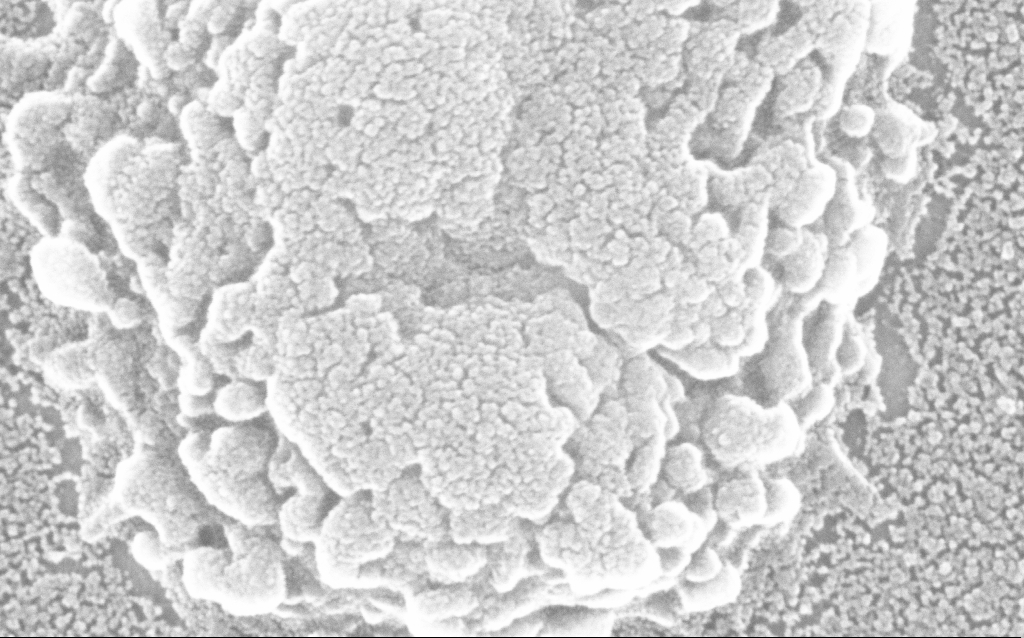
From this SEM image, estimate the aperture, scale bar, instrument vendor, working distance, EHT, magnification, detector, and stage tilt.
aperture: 30 µm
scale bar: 100 nm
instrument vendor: Zeiss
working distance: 1.8 mm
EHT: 20 kV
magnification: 500 K X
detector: InLens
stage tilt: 0°